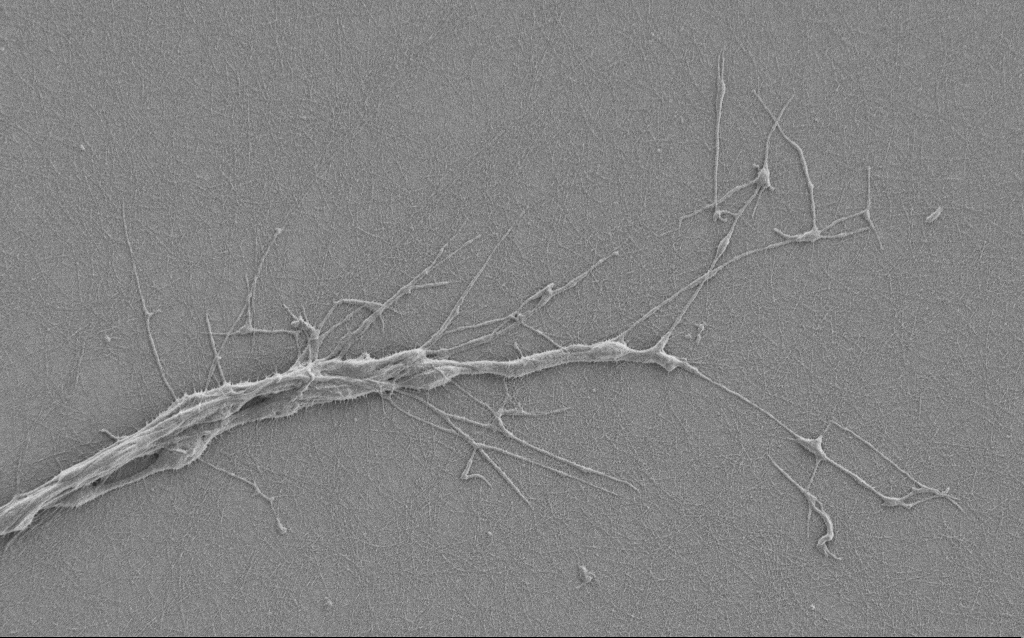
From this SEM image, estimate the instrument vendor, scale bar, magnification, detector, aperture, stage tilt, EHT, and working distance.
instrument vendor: Zeiss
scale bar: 10000 nm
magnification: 6.5 K X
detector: SE2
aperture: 30 µm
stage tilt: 0°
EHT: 1 kV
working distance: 6 mm